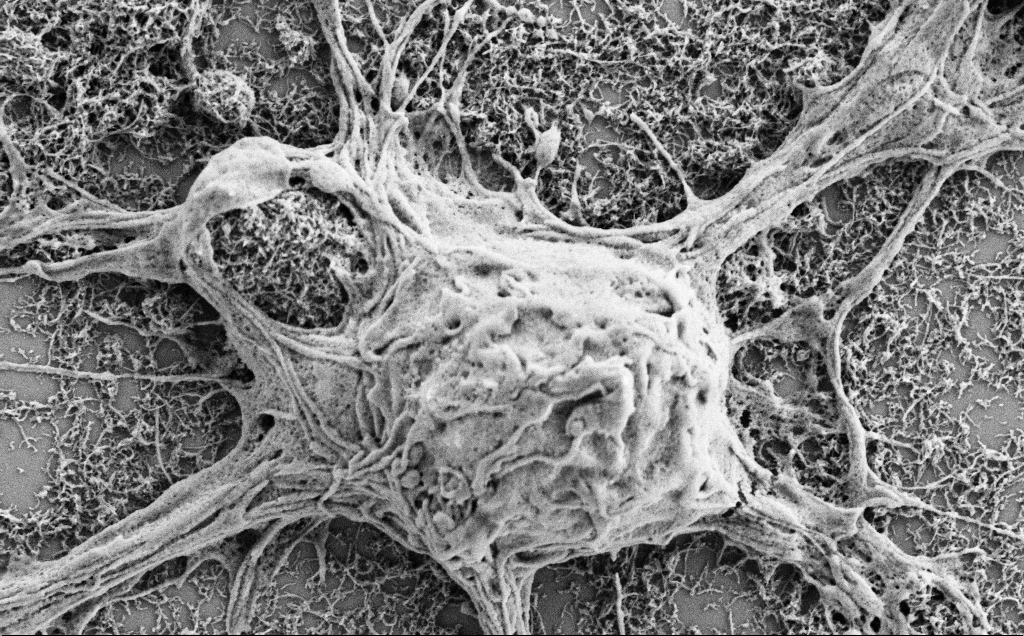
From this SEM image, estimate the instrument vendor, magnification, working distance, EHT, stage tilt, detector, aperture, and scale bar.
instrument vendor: Zeiss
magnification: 20 K X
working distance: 7 mm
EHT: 2 kV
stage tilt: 0°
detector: SE2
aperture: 30 µm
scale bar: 2000 nm